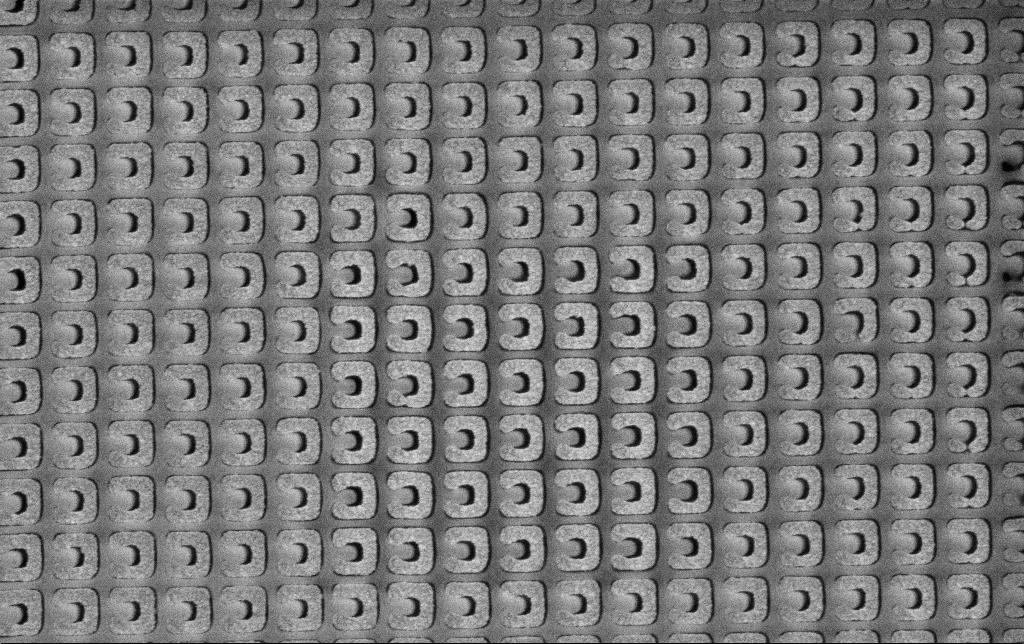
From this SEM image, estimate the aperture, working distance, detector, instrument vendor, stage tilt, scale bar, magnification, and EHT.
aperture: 30 µm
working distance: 4.2 mm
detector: SE2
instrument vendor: Zeiss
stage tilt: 0°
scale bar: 1000 nm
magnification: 44.51 K X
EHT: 3 kV